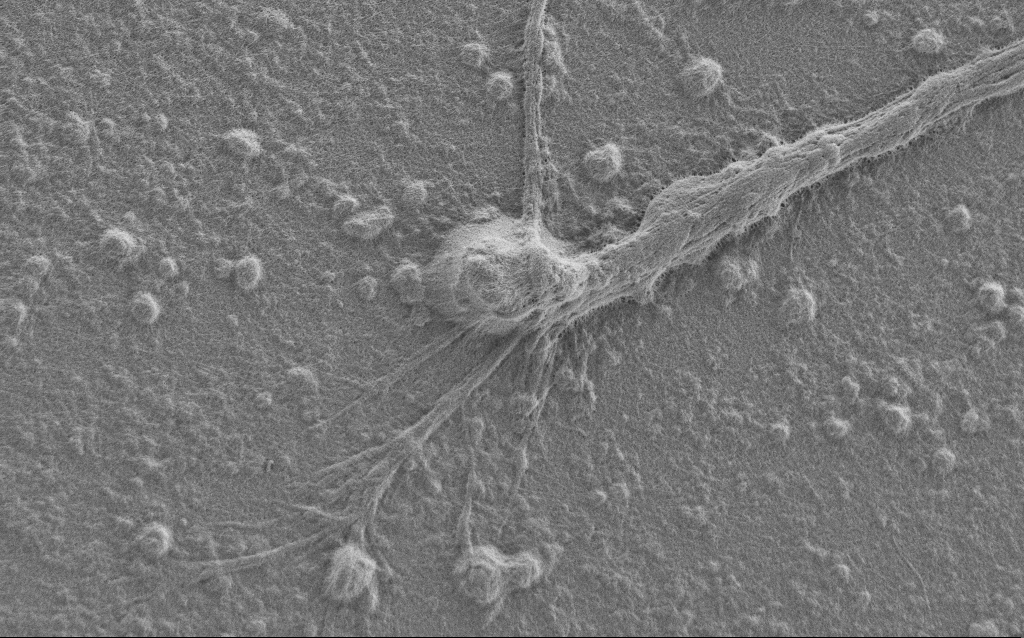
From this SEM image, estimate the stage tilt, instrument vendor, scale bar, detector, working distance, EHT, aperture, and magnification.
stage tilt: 0°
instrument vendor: Zeiss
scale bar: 10000 nm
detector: SE2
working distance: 6 mm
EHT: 1 kV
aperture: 30 µm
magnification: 6.5 K X